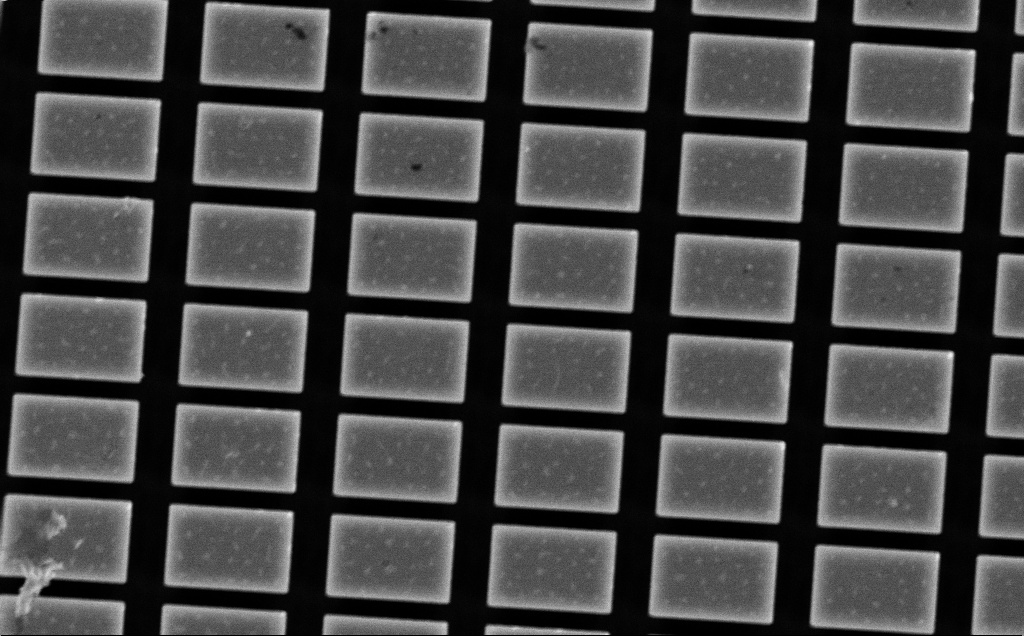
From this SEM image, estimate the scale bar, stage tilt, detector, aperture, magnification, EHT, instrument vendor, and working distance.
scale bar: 2000 nm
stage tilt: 0°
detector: InLens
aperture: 30 µm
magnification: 7.47 K X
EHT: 10 kV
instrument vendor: Zeiss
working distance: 7 mm